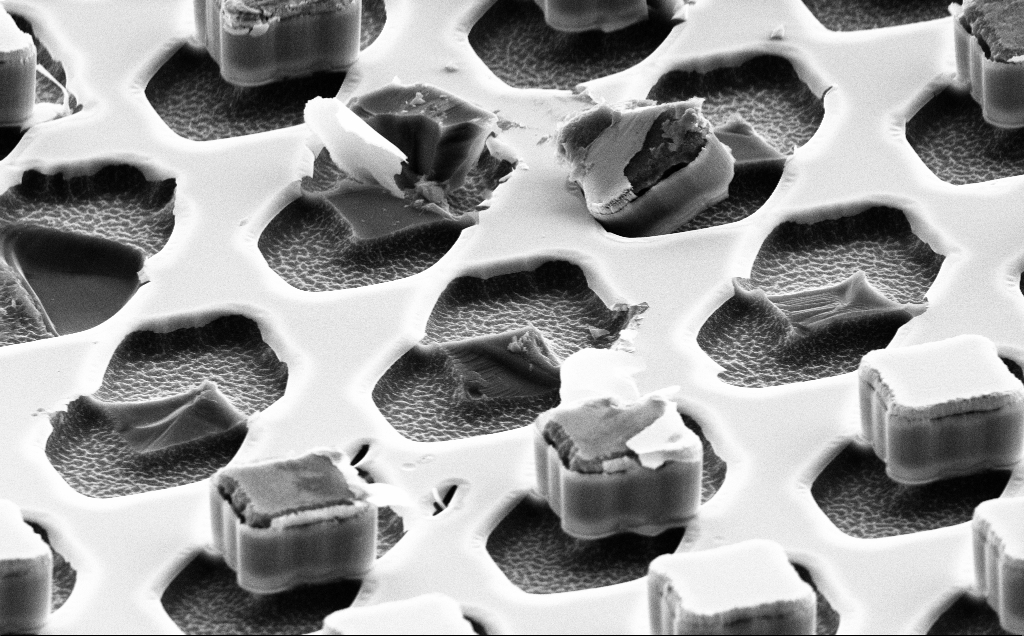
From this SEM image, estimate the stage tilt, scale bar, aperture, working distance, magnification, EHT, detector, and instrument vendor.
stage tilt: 61.7°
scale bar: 10000 nm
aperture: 30 µm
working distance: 12 mm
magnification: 6.3 K X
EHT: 10 kV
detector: SE2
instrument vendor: Zeiss